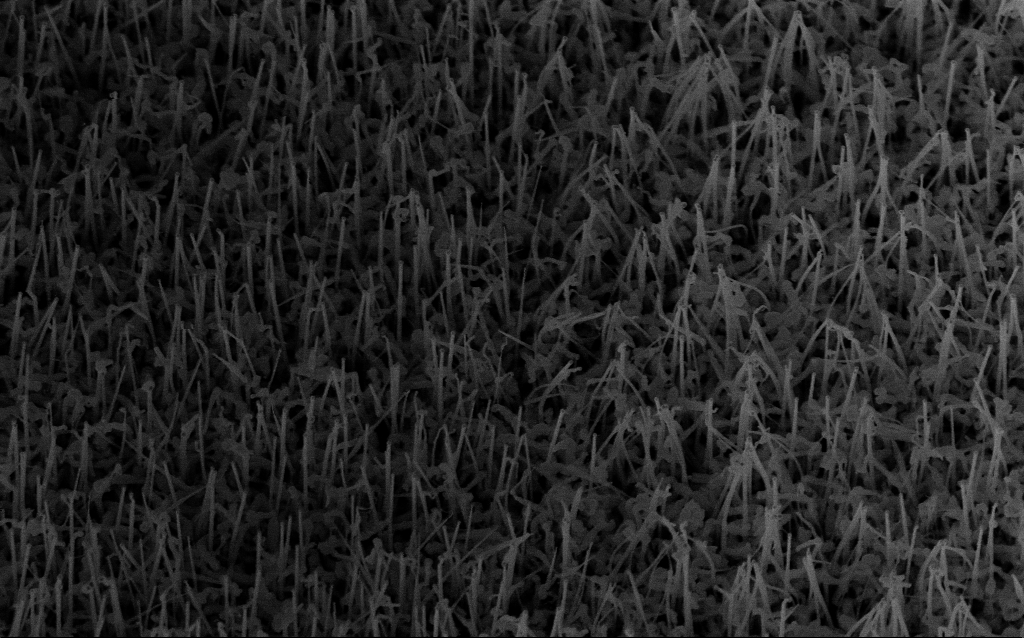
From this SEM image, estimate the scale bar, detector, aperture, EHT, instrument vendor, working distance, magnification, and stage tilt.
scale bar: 1000 nm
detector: InLens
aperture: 30 µm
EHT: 10 kV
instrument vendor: Zeiss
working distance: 7.3 mm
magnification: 44.2 K X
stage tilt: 35°